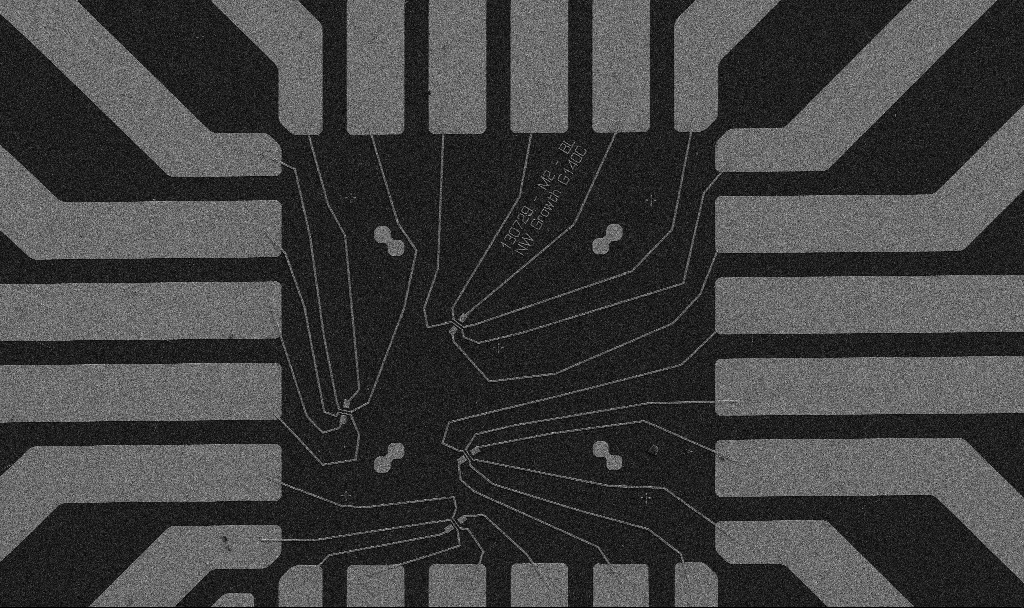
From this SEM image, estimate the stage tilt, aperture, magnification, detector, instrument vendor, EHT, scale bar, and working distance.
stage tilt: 0°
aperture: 30 µm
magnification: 1 K X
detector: SE2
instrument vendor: Zeiss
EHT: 5 kV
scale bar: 20000 nm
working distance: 10.7 mm